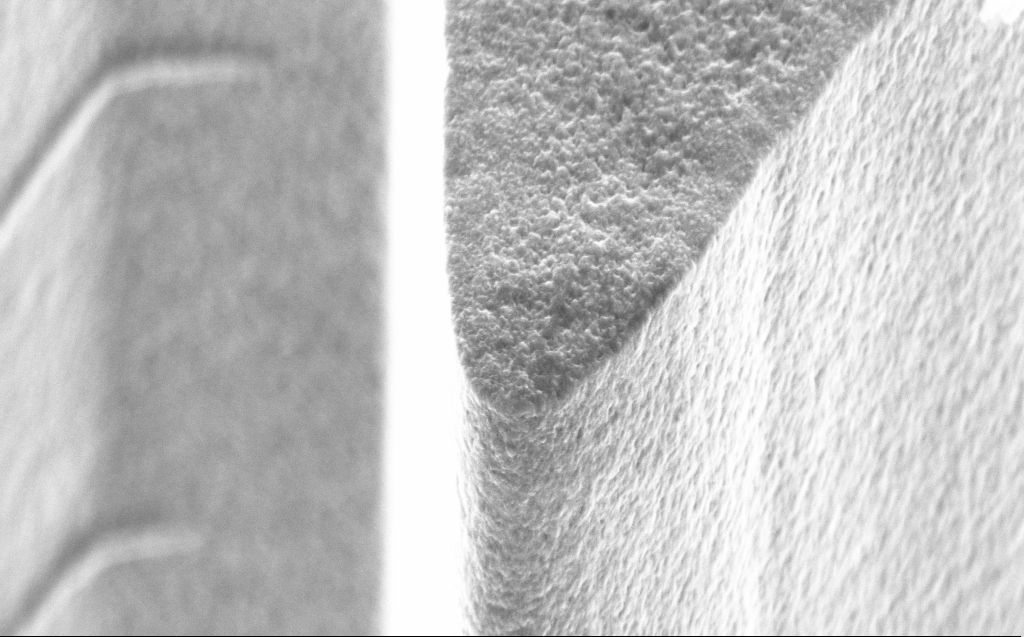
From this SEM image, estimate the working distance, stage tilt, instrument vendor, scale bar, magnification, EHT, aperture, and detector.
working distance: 5 mm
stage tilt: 45°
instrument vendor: Zeiss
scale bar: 1000 nm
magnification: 51.22 K X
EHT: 5 kV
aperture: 30 µm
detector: InLens